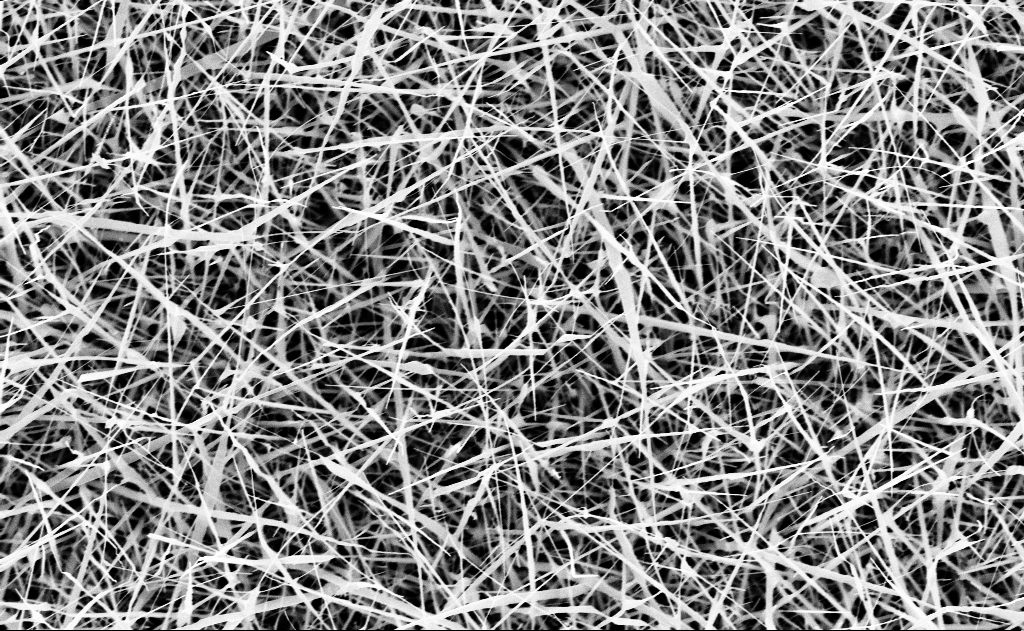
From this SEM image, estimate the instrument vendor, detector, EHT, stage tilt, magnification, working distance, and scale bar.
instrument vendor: Zeiss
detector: InLens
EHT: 10 kV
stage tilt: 0°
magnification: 20 K X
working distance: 15 mm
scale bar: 1000 nm